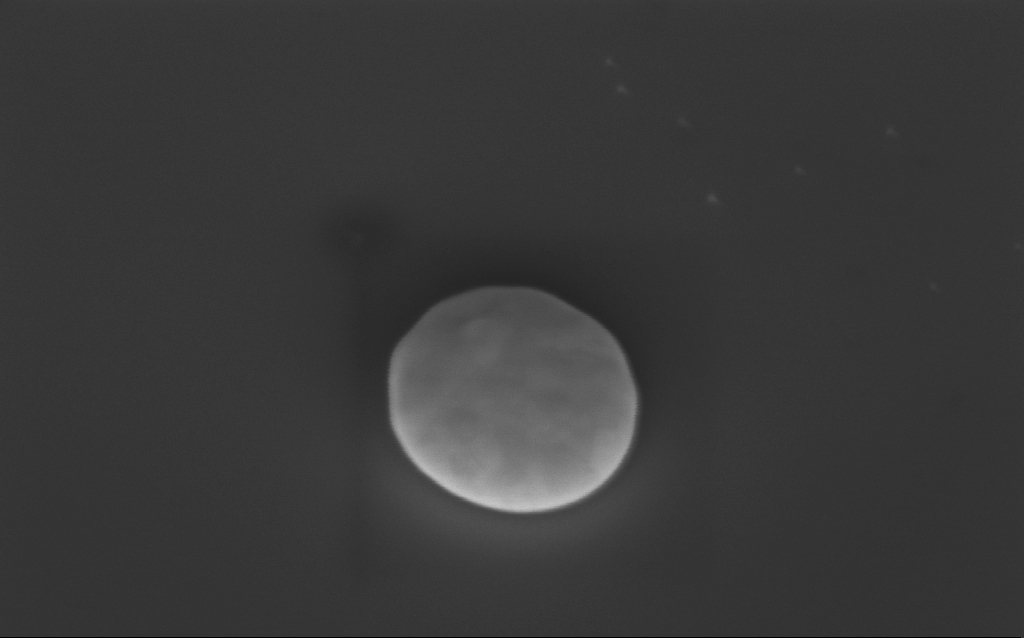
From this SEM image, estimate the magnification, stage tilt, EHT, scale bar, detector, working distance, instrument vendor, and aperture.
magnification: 110.04 K X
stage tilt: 40°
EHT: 3 kV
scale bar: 200 nm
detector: InLens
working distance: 5 mm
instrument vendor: Zeiss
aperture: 30 µm